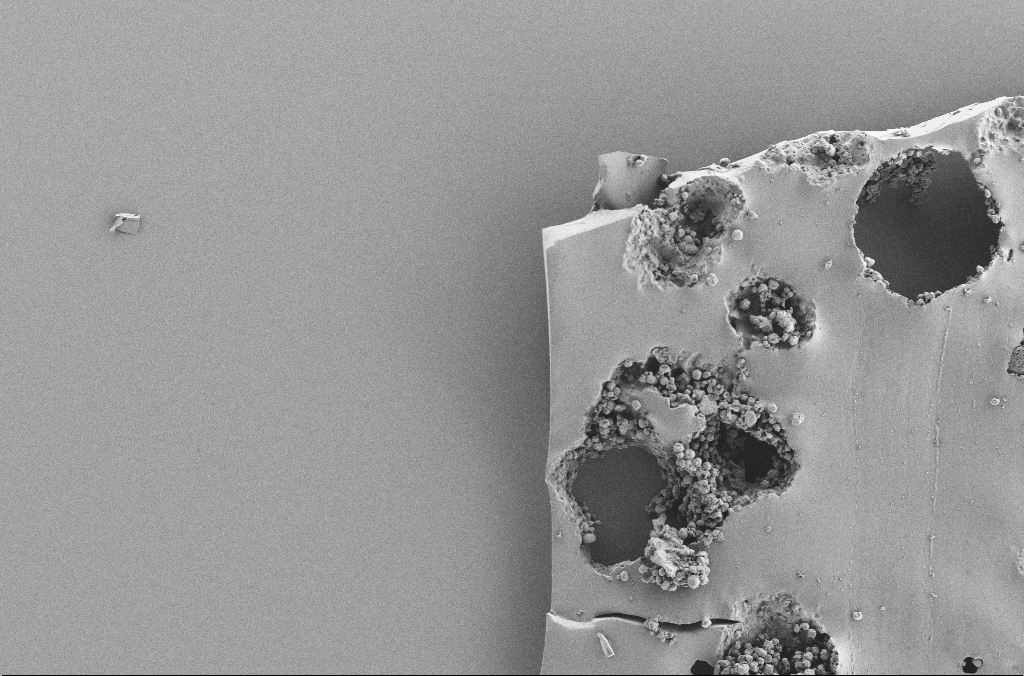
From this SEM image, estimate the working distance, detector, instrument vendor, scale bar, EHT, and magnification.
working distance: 3.7 mm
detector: SE2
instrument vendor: Zeiss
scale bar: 100000 nm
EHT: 2 kV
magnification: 0.25 K X